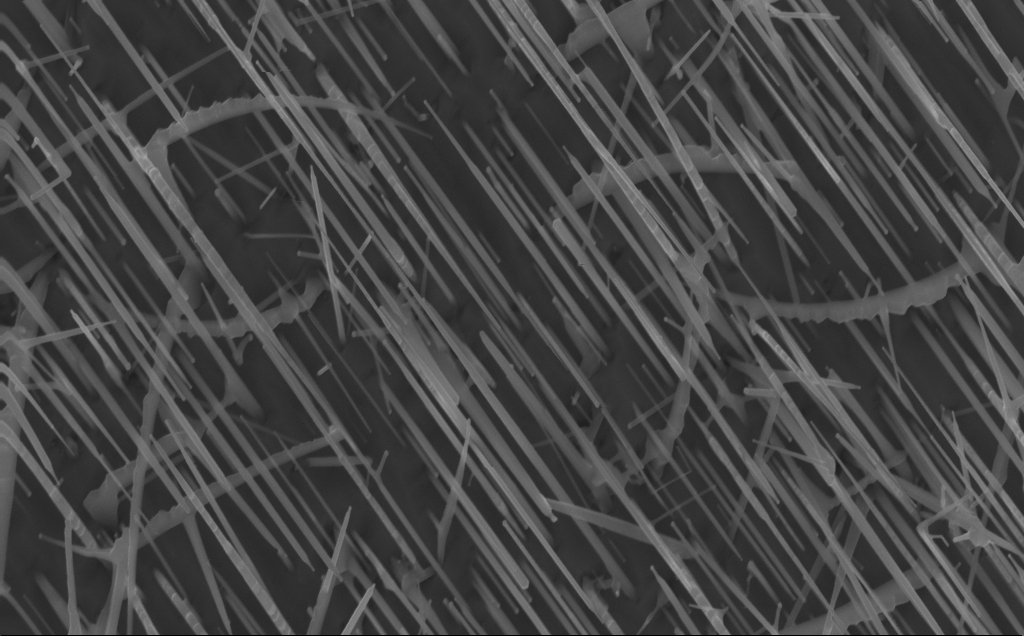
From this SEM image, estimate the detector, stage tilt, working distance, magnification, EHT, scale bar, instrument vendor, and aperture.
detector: InLens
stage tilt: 0°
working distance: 4 mm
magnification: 40 K X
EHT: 10 kV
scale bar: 1000 nm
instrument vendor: Zeiss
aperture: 30 µm